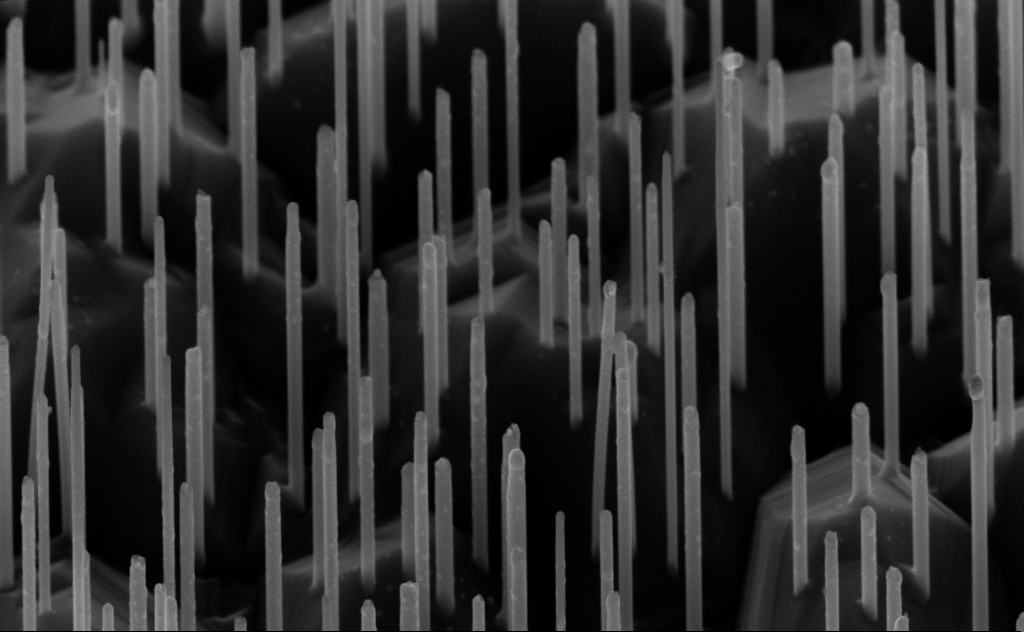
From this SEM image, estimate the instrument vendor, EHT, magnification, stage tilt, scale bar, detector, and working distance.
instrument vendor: Zeiss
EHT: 10 kV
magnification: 80 K X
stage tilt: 45°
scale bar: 200 nm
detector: InLens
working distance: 6 mm